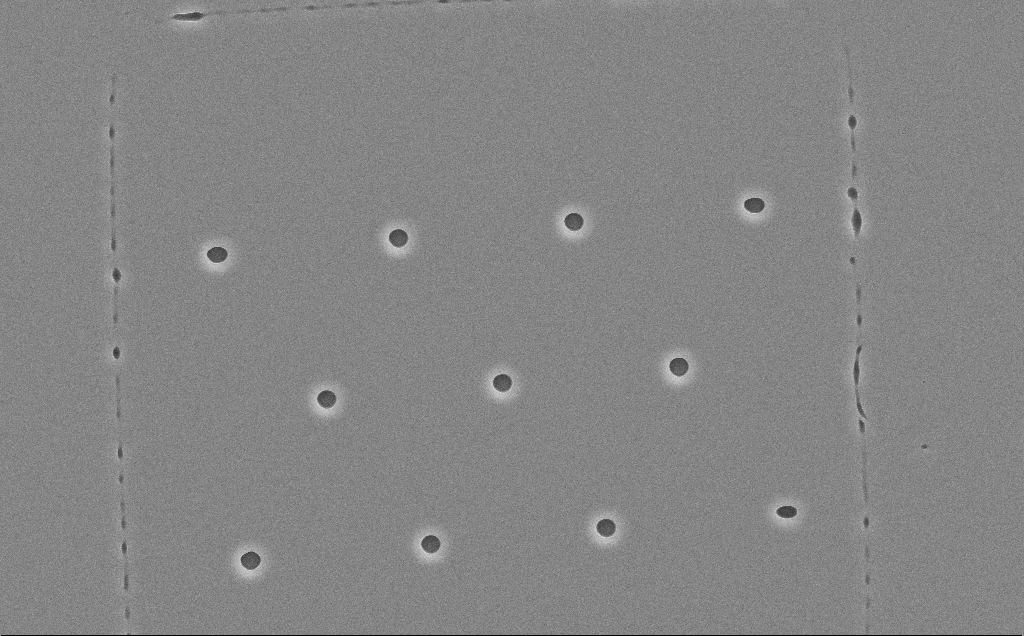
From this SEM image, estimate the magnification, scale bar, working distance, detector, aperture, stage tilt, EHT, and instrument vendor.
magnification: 3.21 K X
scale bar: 10000 nm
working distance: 12 mm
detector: SE2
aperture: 30 µm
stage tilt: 0°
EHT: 10 kV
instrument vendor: Zeiss